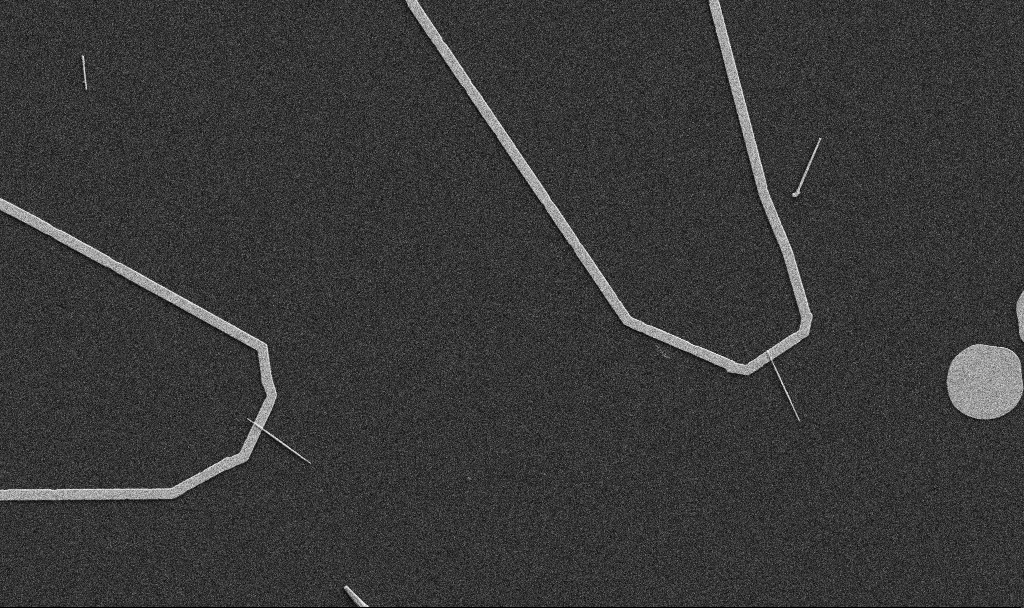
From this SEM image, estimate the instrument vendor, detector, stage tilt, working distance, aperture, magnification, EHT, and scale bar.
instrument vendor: Zeiss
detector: SE2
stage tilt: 0°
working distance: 10.7 mm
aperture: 30 µm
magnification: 5 K X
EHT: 5 kV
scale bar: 10000 nm